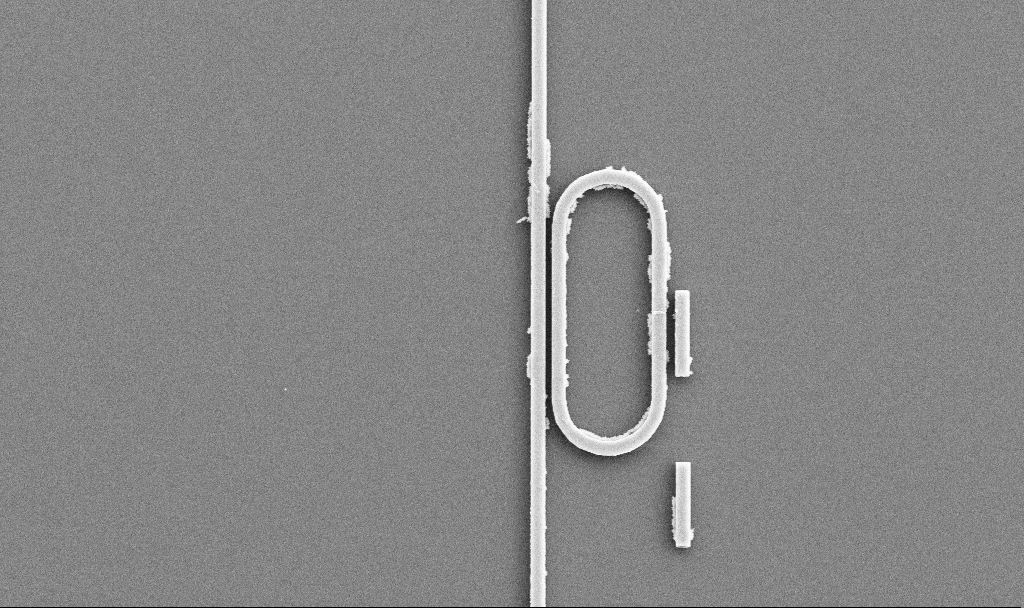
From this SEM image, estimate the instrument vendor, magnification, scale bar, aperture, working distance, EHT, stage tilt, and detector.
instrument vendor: Zeiss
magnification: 10.58 K X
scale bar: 2000 nm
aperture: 30 µm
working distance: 7.5 mm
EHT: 5 kV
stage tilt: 0°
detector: SE2